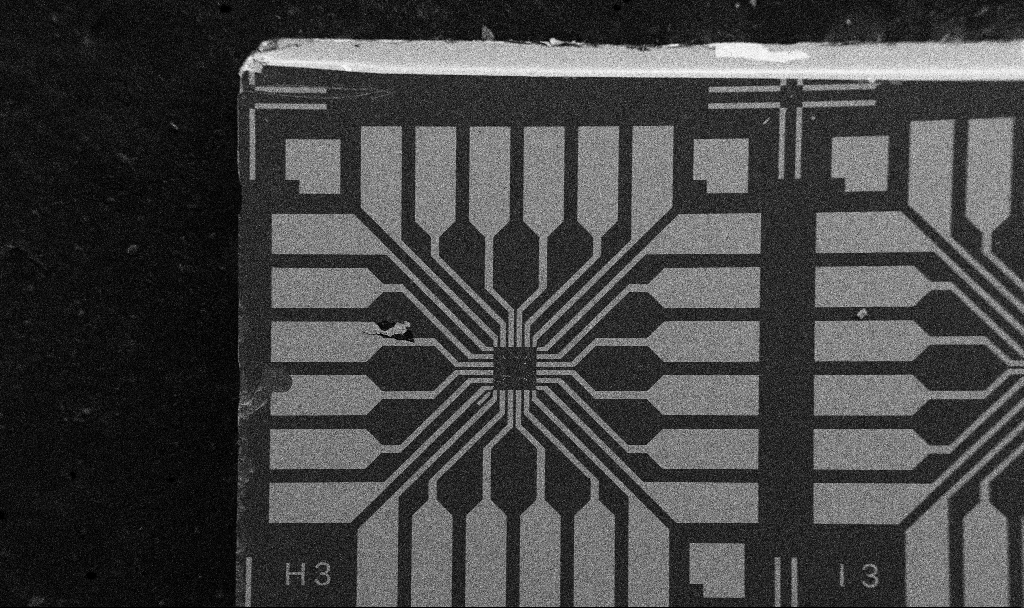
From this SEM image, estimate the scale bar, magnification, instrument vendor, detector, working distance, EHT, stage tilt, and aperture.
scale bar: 200000 nm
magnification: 0.1 K X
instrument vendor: Zeiss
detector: SE2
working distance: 10.7 mm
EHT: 5 kV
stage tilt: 0°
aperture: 30 µm